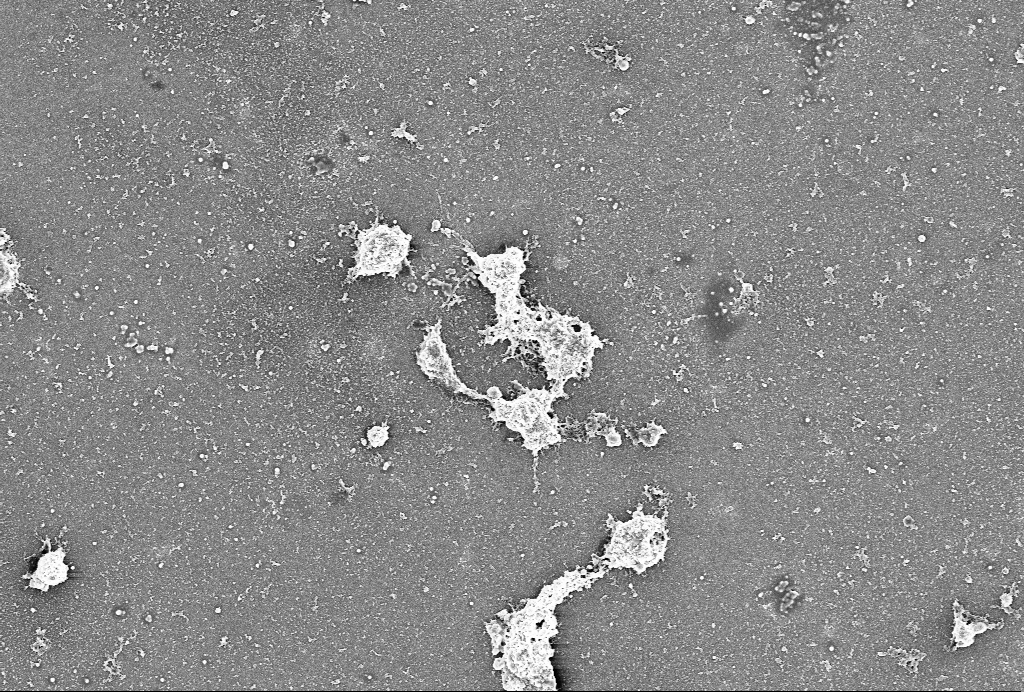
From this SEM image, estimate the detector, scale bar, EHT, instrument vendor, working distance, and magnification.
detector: SE2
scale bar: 10000 nm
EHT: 4 kV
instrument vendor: Zeiss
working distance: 6 mm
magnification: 4 K X